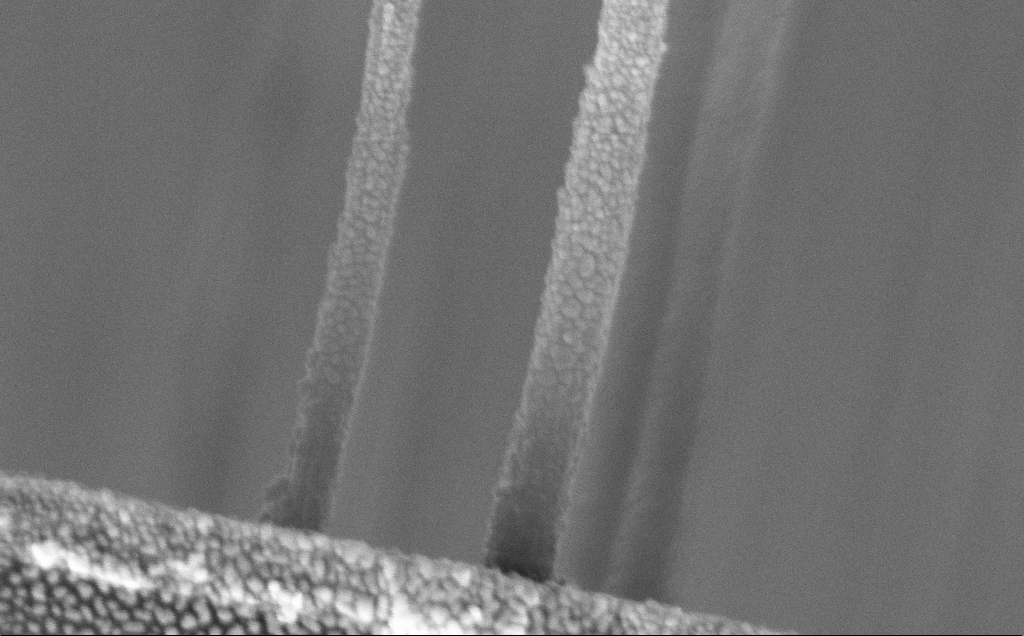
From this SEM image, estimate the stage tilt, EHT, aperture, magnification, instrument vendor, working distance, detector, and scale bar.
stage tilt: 0°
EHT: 5 kV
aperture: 30 µm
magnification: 136.92 K X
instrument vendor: Zeiss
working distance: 11 mm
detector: InLens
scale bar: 200 nm